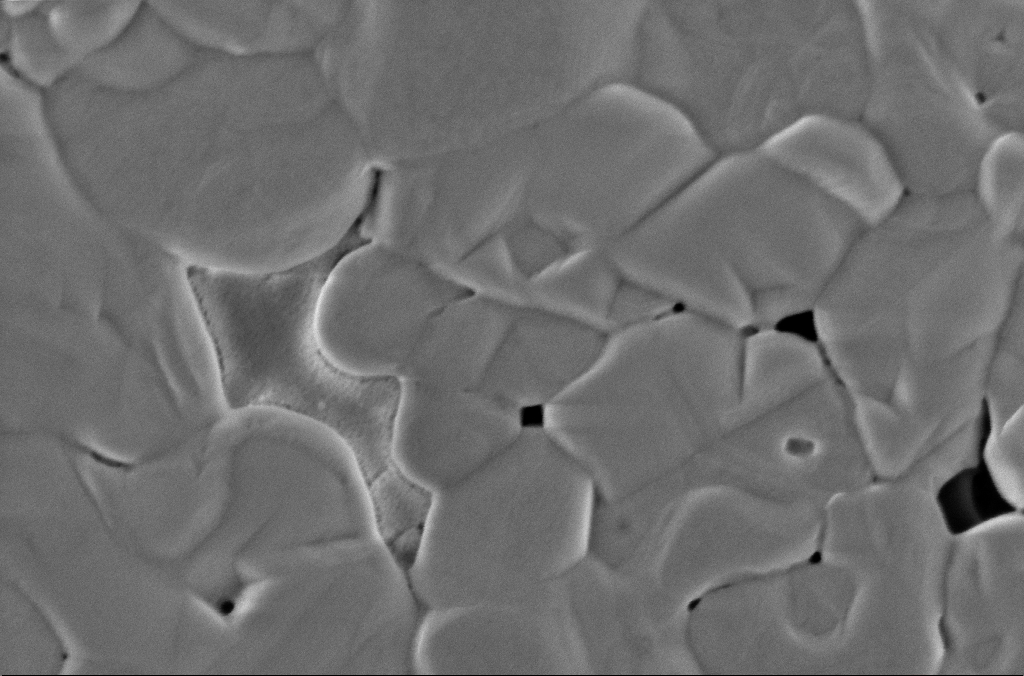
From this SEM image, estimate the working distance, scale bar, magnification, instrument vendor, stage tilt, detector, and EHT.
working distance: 3 mm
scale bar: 200 nm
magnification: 121.42 K X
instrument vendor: Zeiss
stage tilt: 0°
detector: InLens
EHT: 2 kV